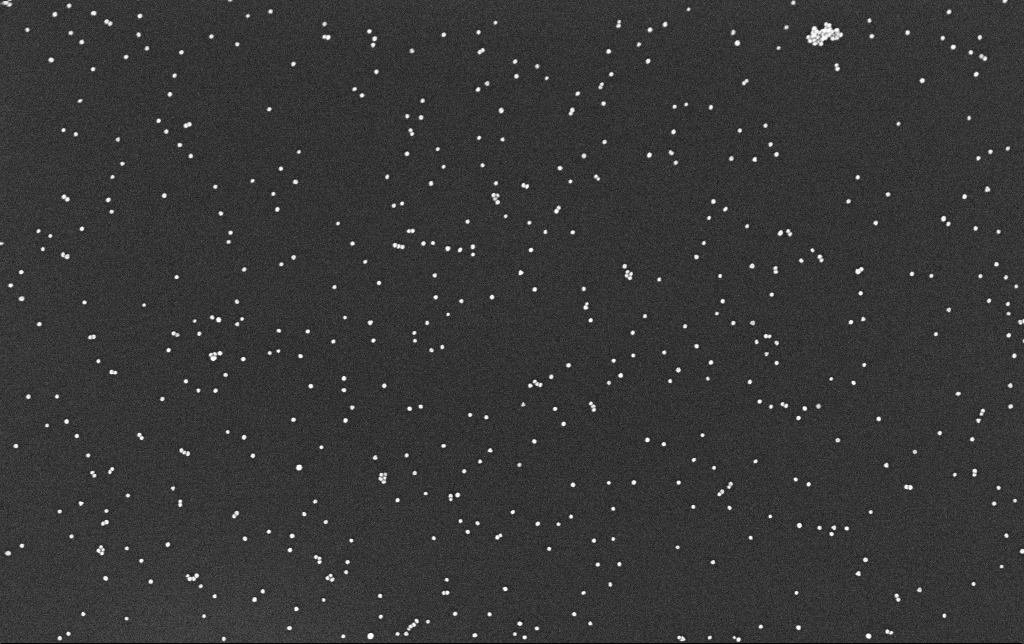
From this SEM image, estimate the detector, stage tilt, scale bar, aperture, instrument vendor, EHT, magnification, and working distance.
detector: InLens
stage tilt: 0°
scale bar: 200 nm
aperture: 30 µm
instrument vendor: Zeiss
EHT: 10 kV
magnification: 100 K X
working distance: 3.4 mm